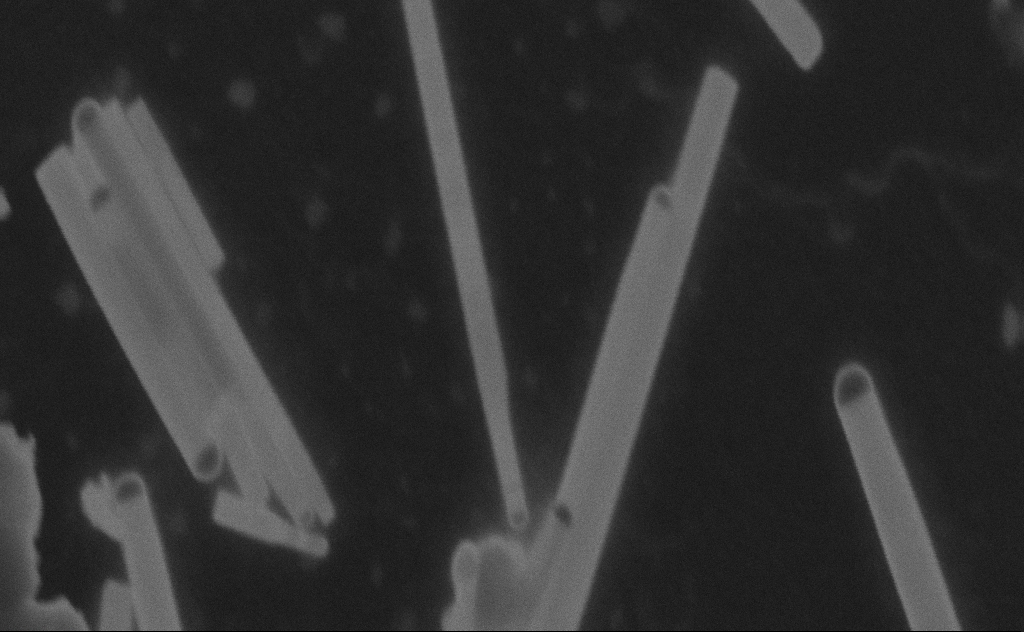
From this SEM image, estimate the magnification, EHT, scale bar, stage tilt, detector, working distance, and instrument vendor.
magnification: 245.69 K X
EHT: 20 kV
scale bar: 200 nm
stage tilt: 0°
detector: SE2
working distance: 9 mm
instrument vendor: Zeiss